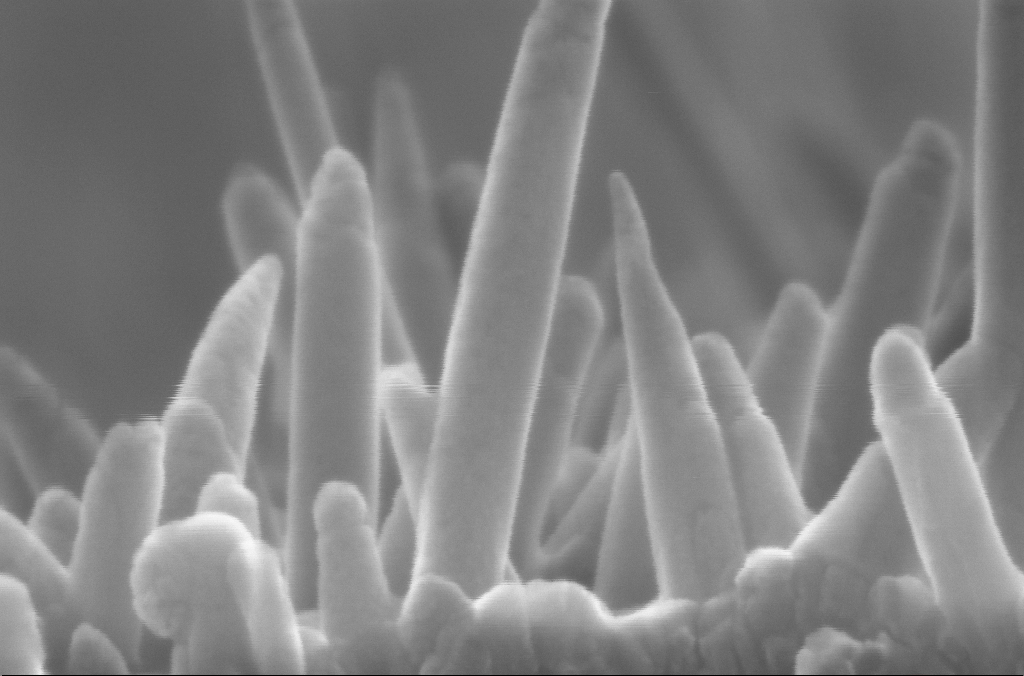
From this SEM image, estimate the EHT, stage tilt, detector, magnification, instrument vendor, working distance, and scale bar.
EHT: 10 kV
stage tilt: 4°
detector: InLens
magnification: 543.47 K X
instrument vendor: Zeiss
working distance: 2.6 mm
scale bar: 100 nm